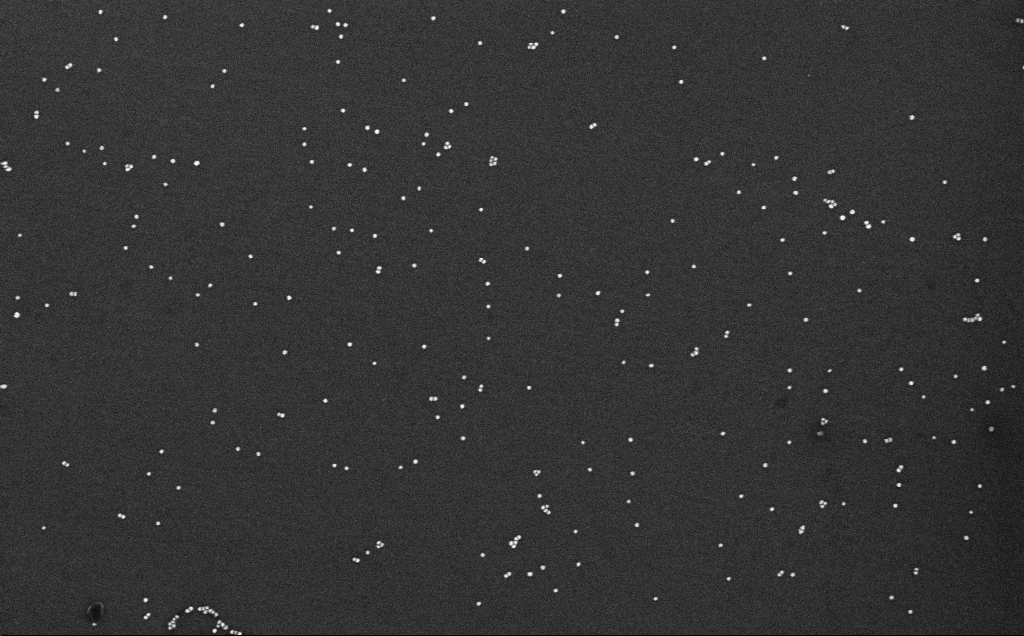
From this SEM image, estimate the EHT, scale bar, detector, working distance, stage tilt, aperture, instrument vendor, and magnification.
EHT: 10 kV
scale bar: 200 nm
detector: InLens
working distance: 6.6 mm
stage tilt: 0°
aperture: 30 µm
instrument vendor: Zeiss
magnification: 100 K X